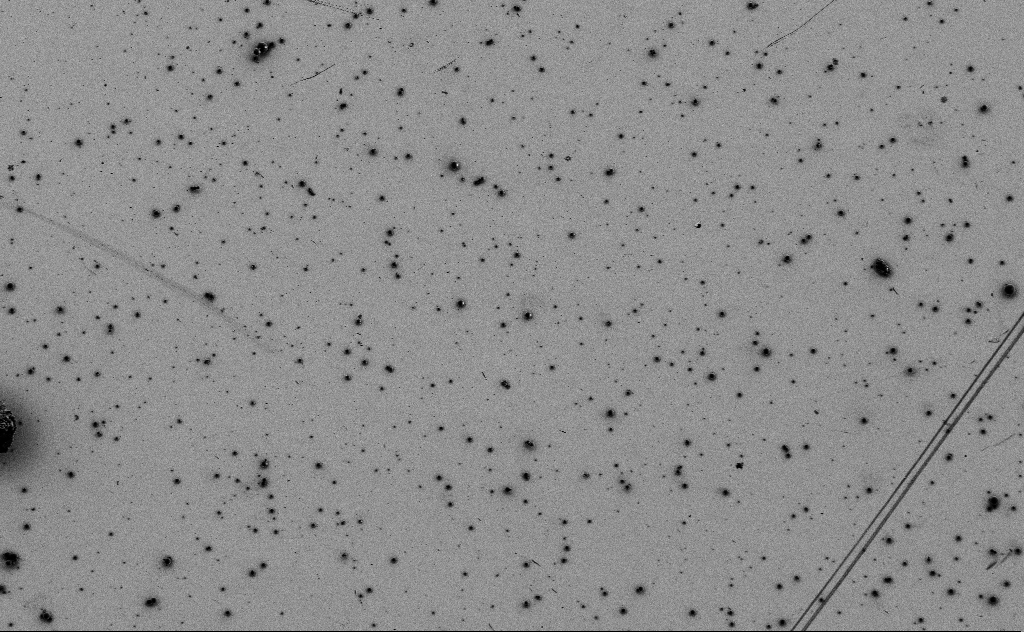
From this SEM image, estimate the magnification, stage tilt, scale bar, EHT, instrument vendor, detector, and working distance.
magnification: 0.963 K X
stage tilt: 0°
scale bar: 20000 nm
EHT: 3 kV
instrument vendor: Zeiss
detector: SE2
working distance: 10 mm